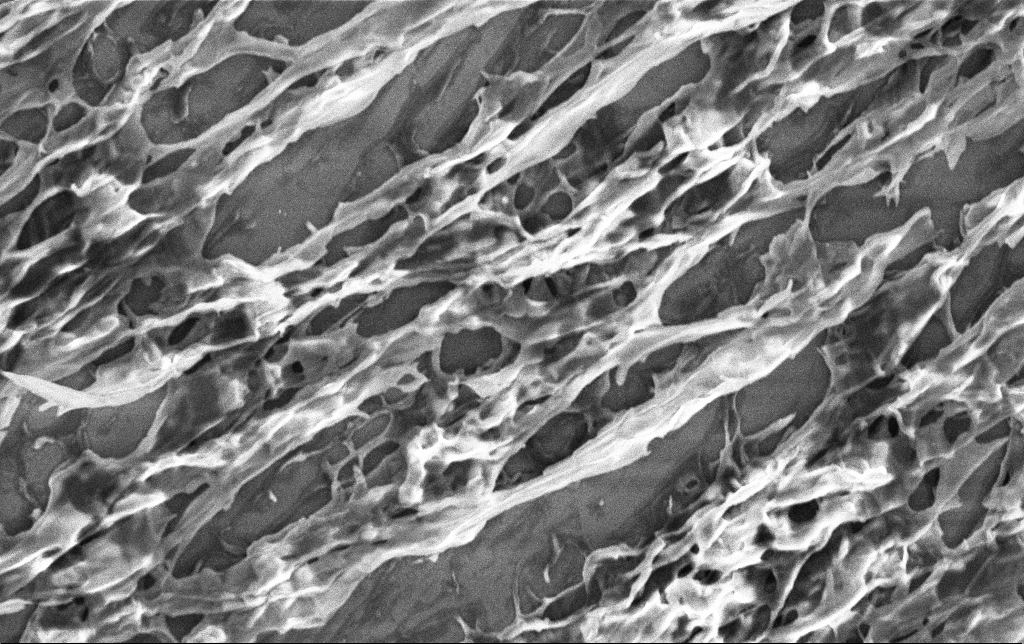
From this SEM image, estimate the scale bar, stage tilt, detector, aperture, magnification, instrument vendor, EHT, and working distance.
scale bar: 2000 nm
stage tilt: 0°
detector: InLens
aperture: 30 µm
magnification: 13.36 K X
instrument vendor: Zeiss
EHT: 3 kV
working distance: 3.2 mm